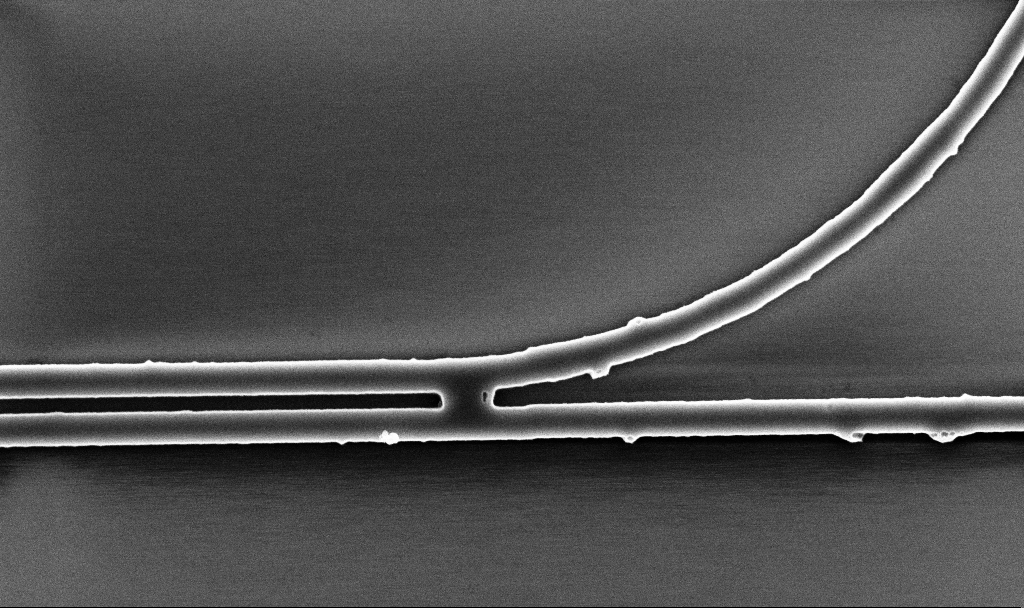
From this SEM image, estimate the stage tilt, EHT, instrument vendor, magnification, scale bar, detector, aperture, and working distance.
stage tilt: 0°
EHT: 5 kV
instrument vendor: Zeiss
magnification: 26.25 K X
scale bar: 2000 nm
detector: InLens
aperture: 30 µm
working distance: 5.2 mm